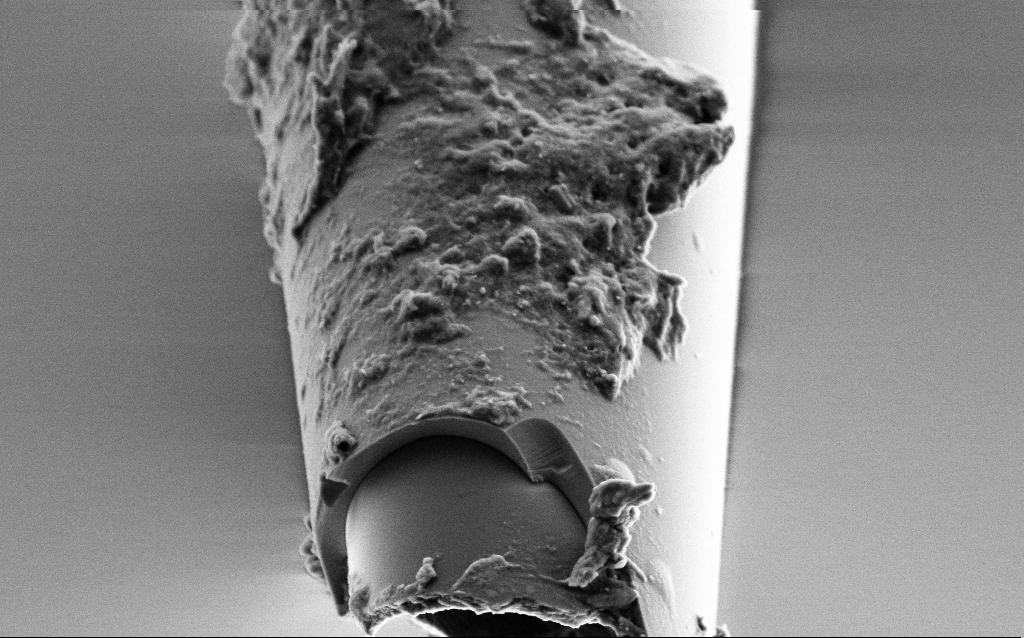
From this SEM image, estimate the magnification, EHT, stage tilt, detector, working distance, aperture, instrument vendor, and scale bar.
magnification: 25 K X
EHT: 2 kV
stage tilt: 45°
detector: SE2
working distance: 6 mm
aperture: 30 µm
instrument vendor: Zeiss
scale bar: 1000 nm